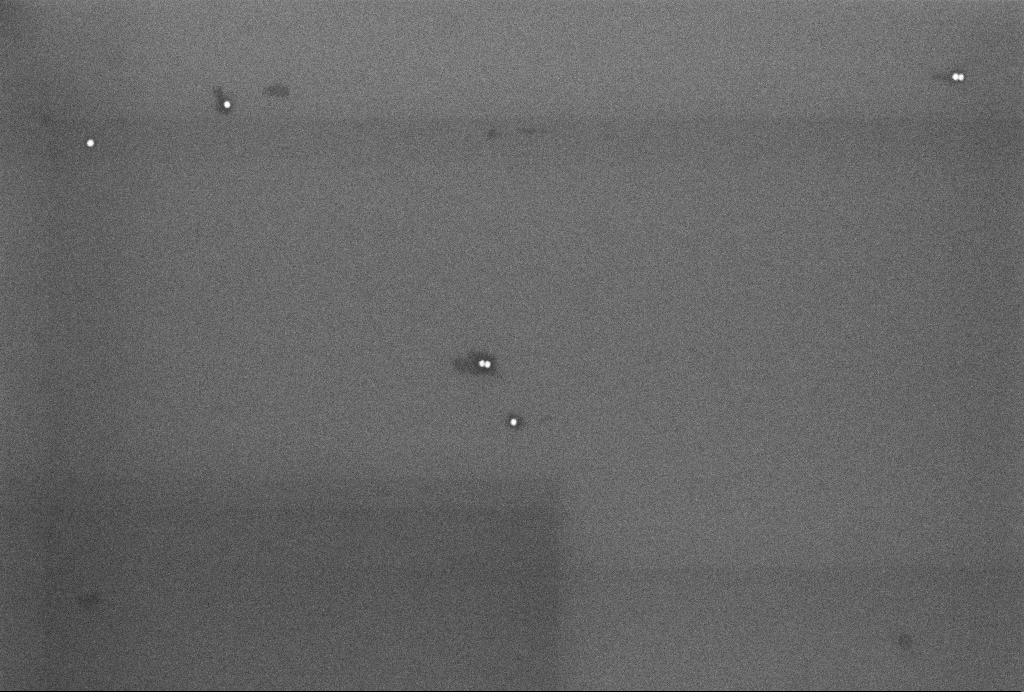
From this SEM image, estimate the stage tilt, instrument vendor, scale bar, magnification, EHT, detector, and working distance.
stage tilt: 0°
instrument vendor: Zeiss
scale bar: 200 nm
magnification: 90 K X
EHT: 4 kV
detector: InLens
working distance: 3.2 mm